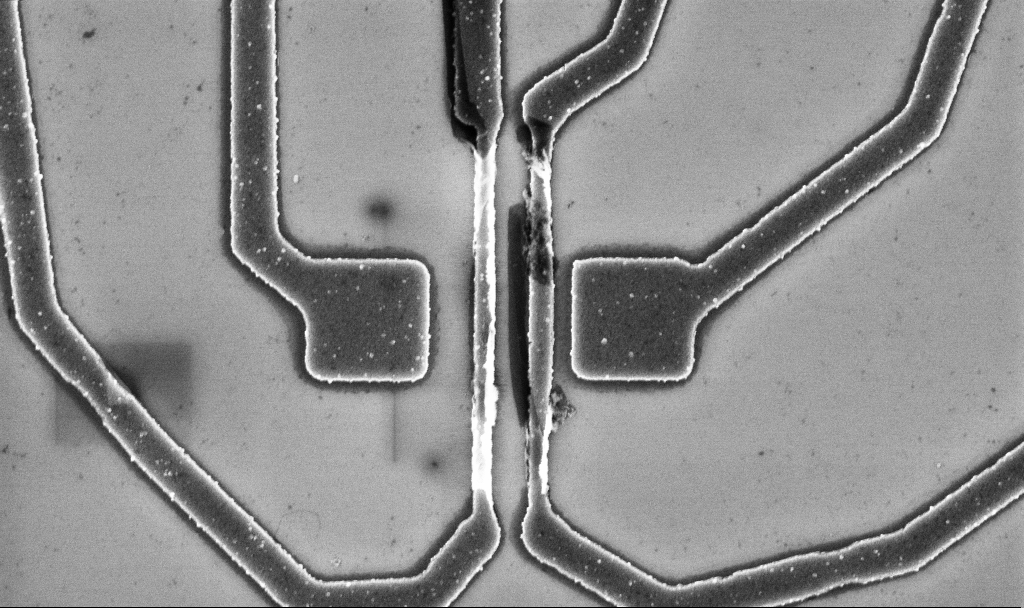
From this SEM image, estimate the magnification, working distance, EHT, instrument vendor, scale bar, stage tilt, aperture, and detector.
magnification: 20 K X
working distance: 10.7 mm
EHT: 5 kV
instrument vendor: Zeiss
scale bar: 2000 nm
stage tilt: -0°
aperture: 30 µm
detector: InLens